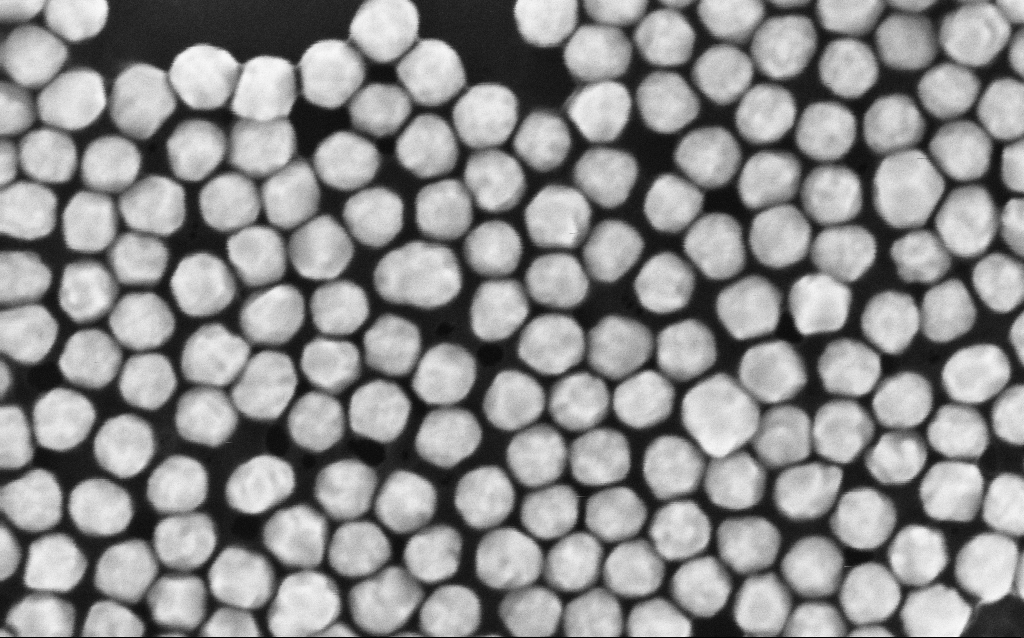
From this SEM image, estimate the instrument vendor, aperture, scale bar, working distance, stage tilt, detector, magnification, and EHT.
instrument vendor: Zeiss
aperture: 30 µm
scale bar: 100 nm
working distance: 3.1 mm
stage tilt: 0°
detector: InLens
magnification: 391.37 K X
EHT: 10 kV